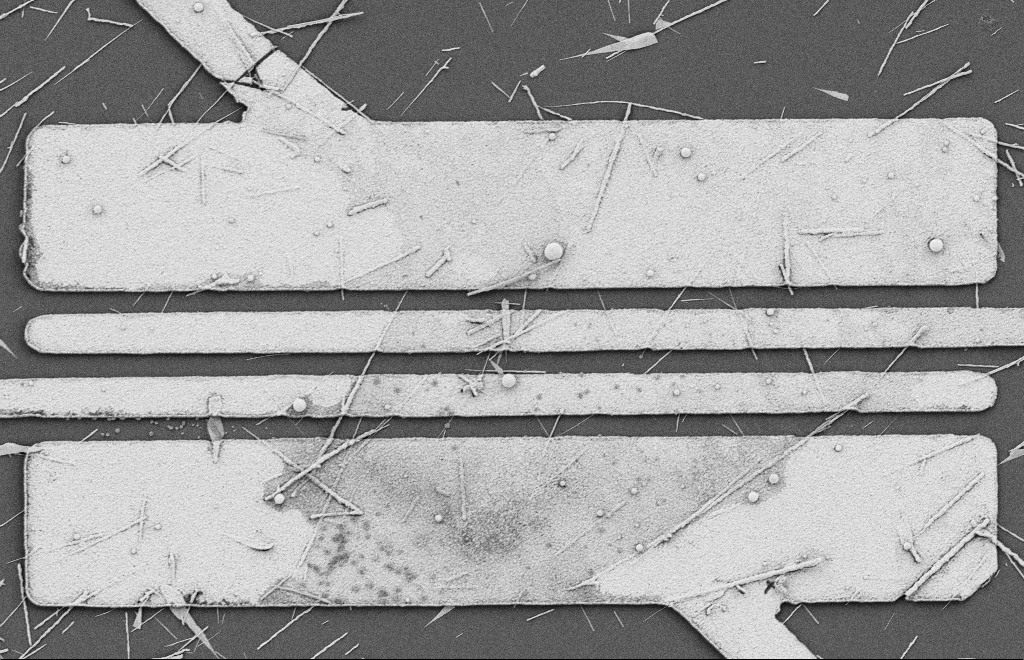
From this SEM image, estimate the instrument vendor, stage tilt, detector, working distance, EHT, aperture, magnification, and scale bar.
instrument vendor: Zeiss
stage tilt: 0°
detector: SE2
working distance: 10 mm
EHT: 2 kV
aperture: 20 µm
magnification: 5.82 K X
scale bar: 2000 nm